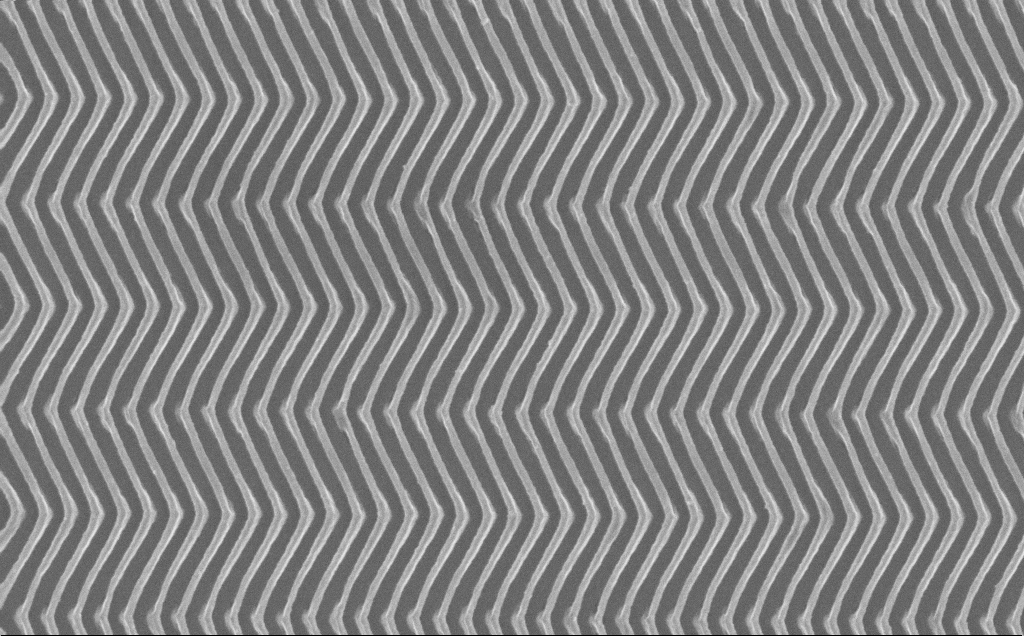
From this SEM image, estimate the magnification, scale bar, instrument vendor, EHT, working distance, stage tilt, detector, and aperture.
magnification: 38.35 K X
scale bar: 1000 nm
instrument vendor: Zeiss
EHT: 10 kV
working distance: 7 mm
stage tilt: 0°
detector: InLens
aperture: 30 µm